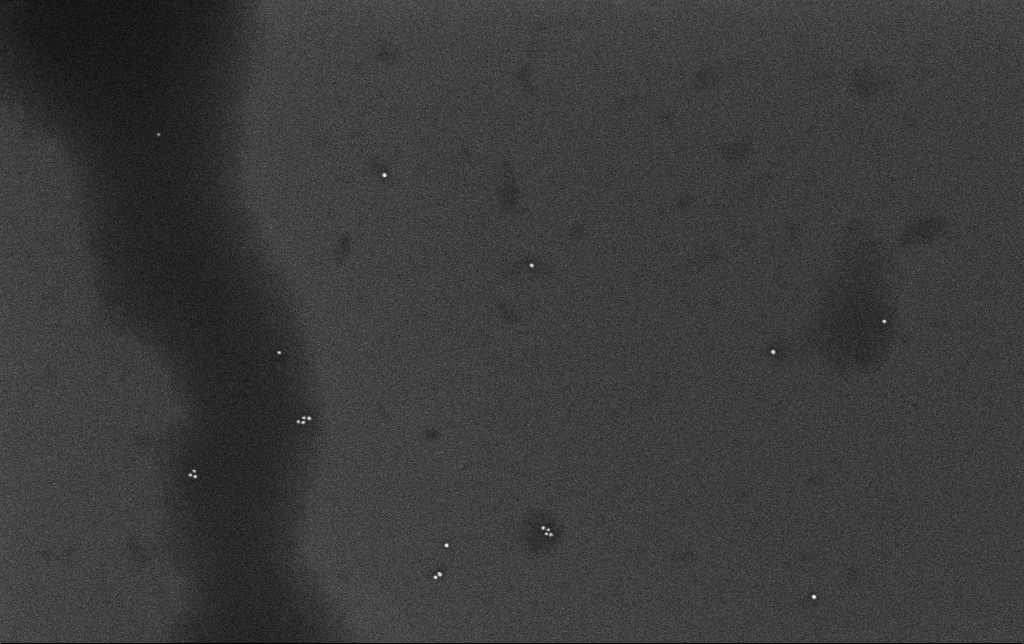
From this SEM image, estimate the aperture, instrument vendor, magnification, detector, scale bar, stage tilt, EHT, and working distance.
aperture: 30 µm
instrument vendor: Zeiss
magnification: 100 K X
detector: InLens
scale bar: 200 nm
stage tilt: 0°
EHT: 10 kV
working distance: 3.4 mm